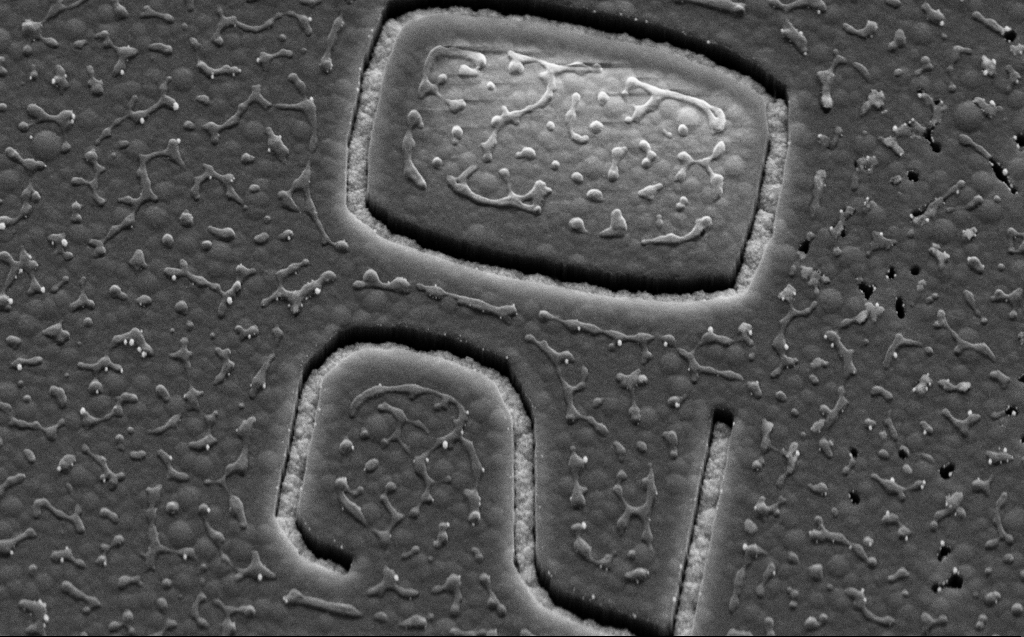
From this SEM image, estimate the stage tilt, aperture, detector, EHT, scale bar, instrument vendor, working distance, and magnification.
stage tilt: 45°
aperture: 30 µm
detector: SE2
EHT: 5 kV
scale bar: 2000 nm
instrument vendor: Zeiss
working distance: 9 mm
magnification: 32.42 K X